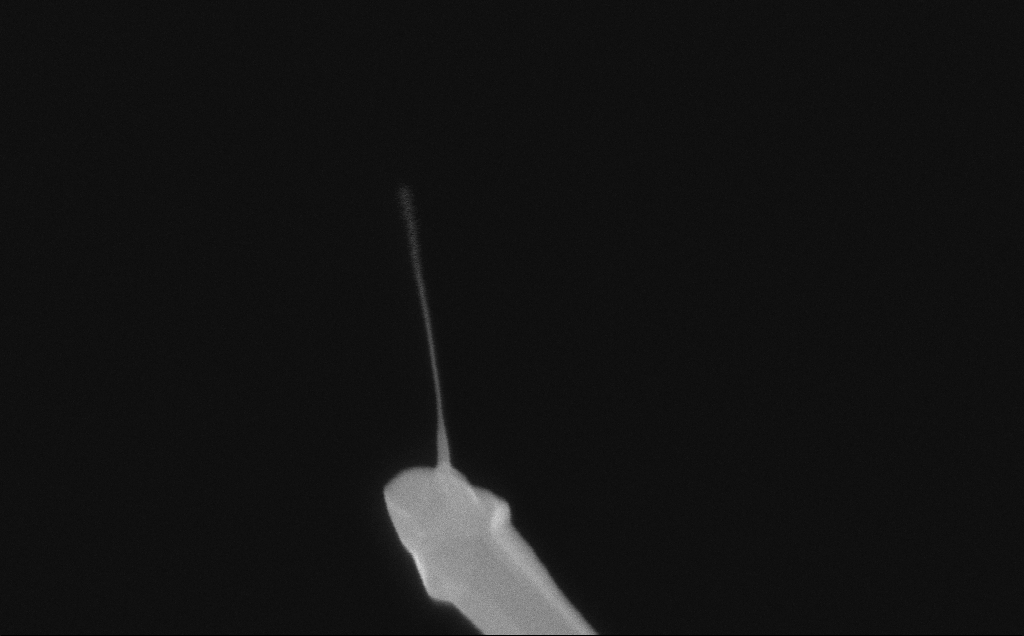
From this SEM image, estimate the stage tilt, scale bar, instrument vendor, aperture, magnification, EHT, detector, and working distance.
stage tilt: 0°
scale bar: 200 nm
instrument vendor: Zeiss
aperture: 30 µm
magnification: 189.64 K X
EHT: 10 kV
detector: InLens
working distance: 6 mm